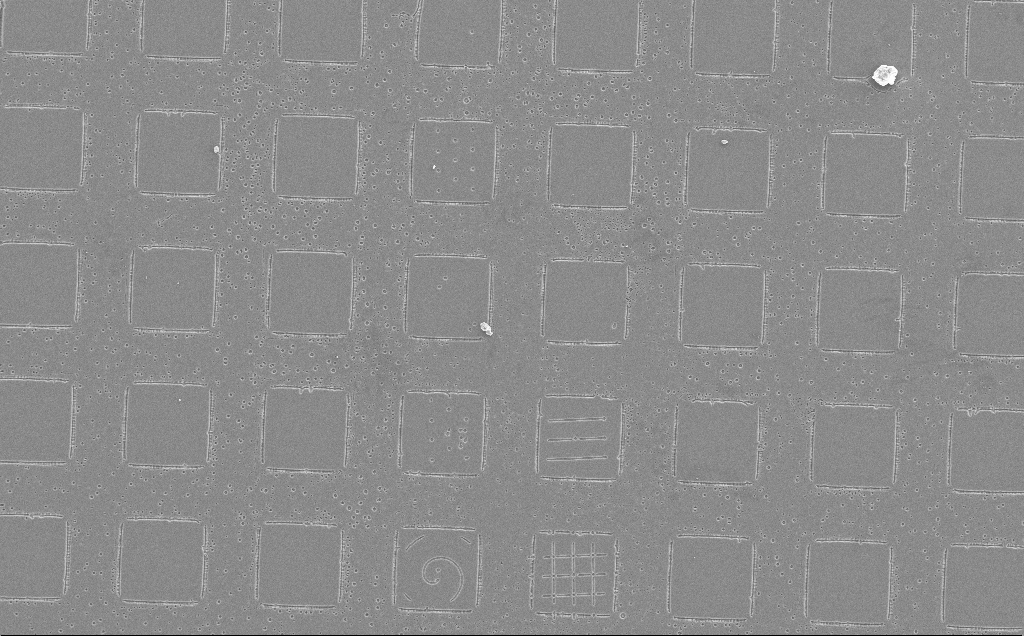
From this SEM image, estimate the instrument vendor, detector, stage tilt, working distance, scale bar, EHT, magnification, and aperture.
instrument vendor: Zeiss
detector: SE2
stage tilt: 0°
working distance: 13 mm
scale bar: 100000 nm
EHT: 10 kV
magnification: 0.357 K X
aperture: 30 µm